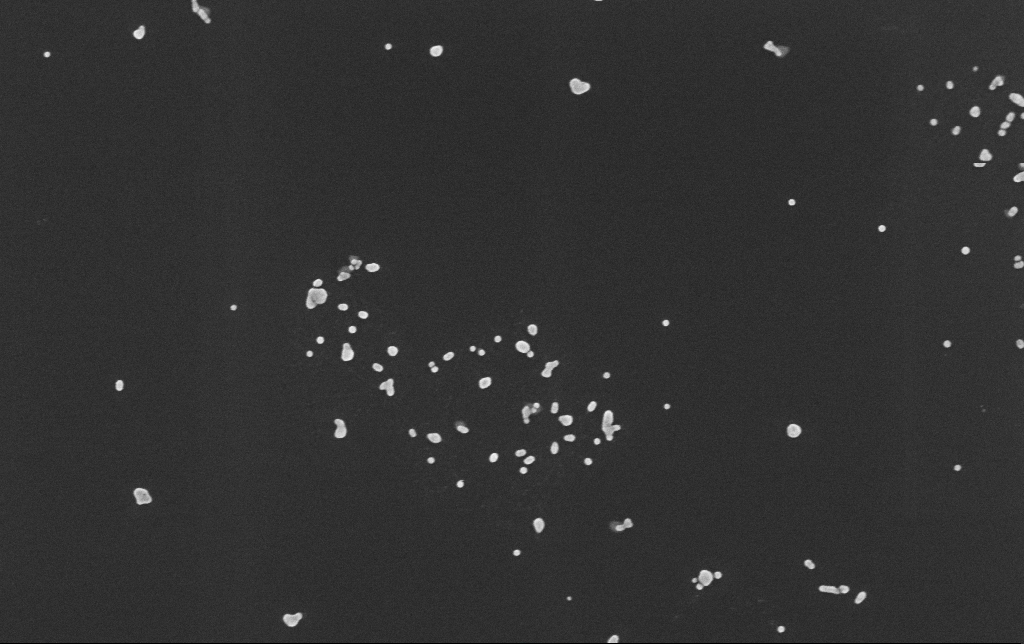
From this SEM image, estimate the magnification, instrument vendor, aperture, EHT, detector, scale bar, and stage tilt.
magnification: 100 K X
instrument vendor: Zeiss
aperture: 30 µm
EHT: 10 kV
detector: InLens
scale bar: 200 nm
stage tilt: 0°